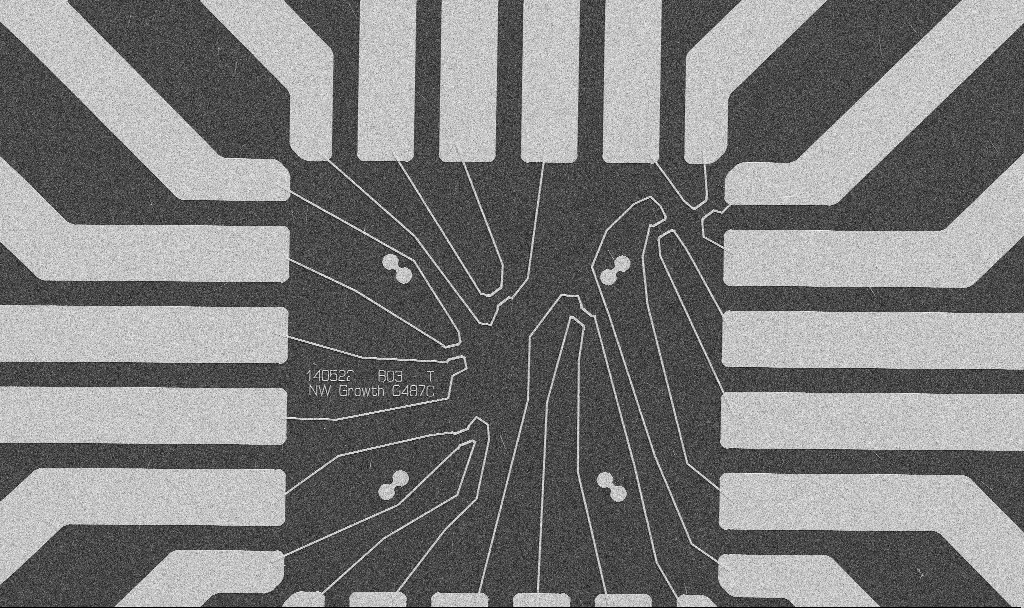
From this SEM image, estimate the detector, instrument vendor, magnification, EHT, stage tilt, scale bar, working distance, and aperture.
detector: SE2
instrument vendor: Zeiss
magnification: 1 K X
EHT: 5 kV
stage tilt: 0°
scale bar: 20000 nm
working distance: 10.7 mm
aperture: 30 µm